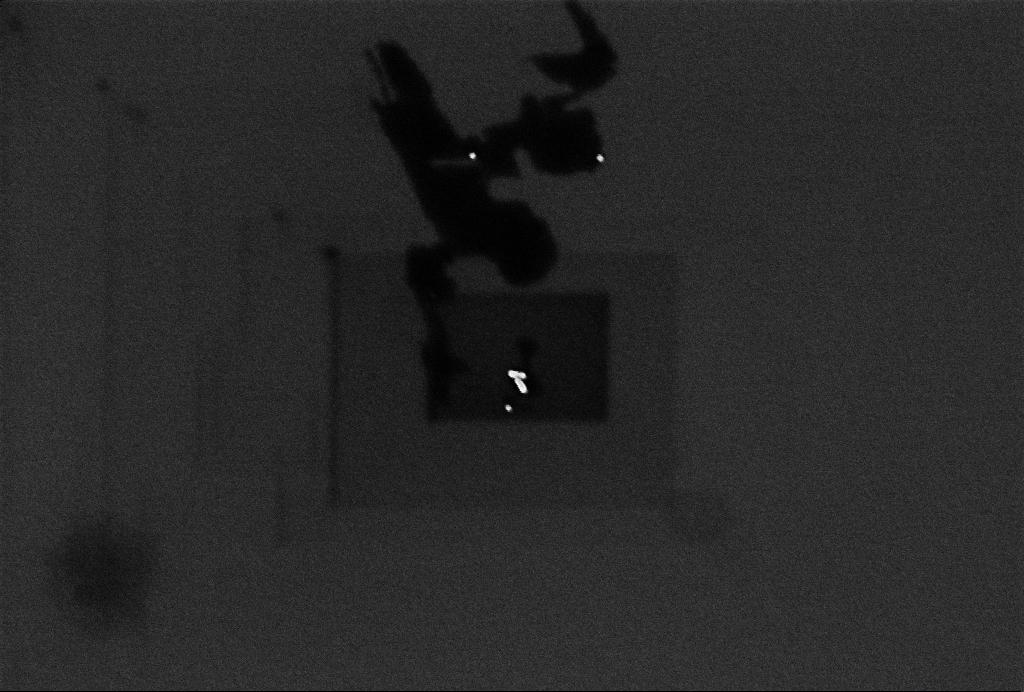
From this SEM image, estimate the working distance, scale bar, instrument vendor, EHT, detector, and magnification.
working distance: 3.3 mm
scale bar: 200 nm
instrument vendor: Zeiss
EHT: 1 kV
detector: InLens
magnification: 80.02 K X